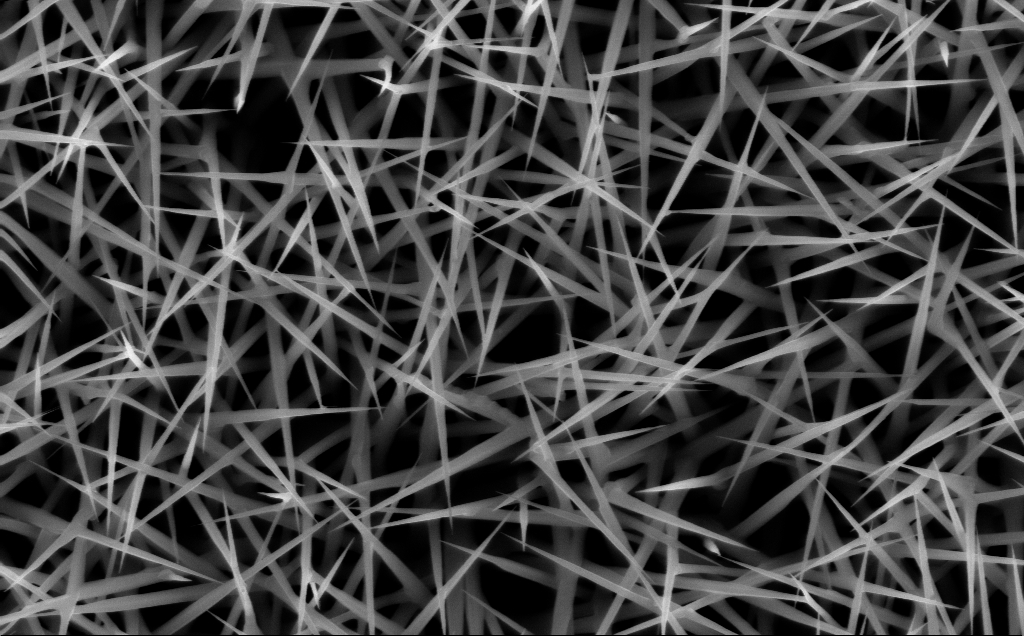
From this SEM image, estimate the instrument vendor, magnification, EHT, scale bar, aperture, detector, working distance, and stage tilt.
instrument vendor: Zeiss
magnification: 40 K X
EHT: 10 kV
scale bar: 1000 nm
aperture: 30 µm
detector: InLens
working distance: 7 mm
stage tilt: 0°